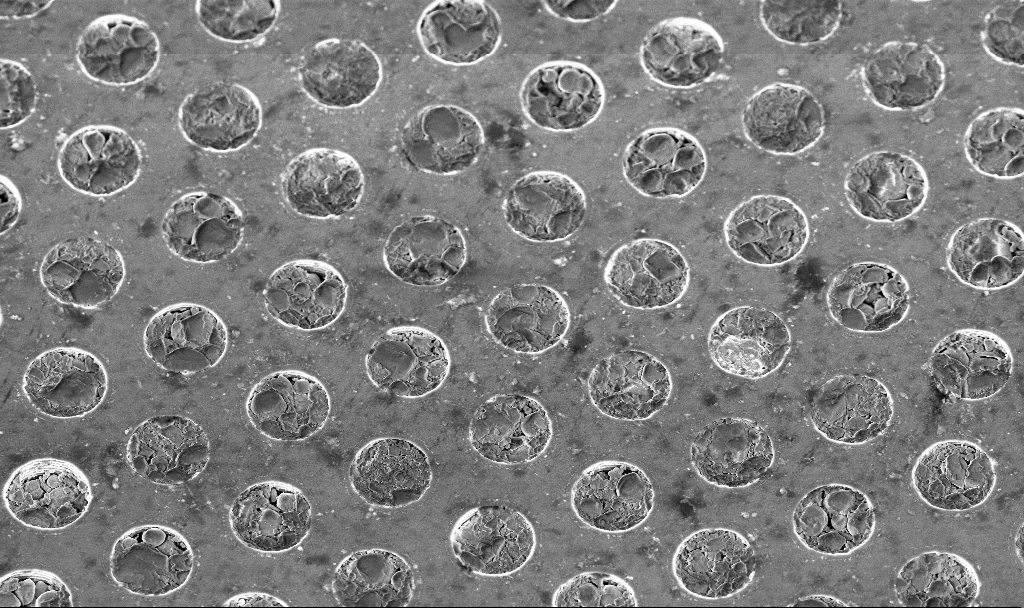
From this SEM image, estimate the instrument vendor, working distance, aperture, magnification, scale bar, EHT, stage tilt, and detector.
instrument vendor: Zeiss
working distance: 4.6 mm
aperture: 30 µm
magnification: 7.92 K X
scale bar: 2000 nm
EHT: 3 kV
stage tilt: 30°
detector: InLens